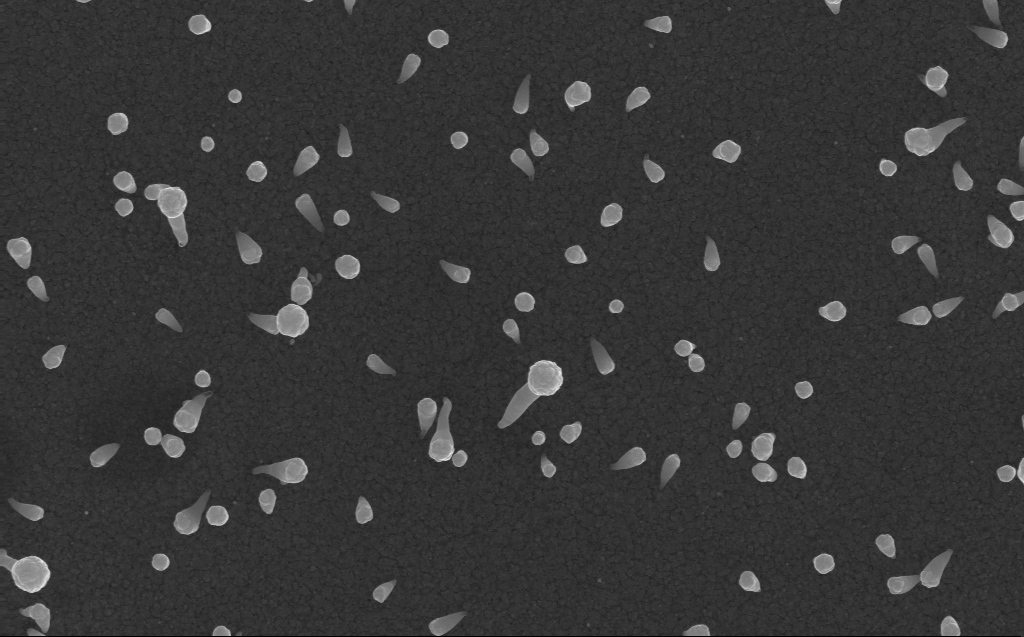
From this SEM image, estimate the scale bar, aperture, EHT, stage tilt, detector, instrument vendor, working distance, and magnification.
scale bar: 1000 nm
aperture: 30 µm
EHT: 10 kV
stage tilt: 0°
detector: InLens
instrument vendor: Zeiss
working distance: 3 mm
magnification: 50 K X